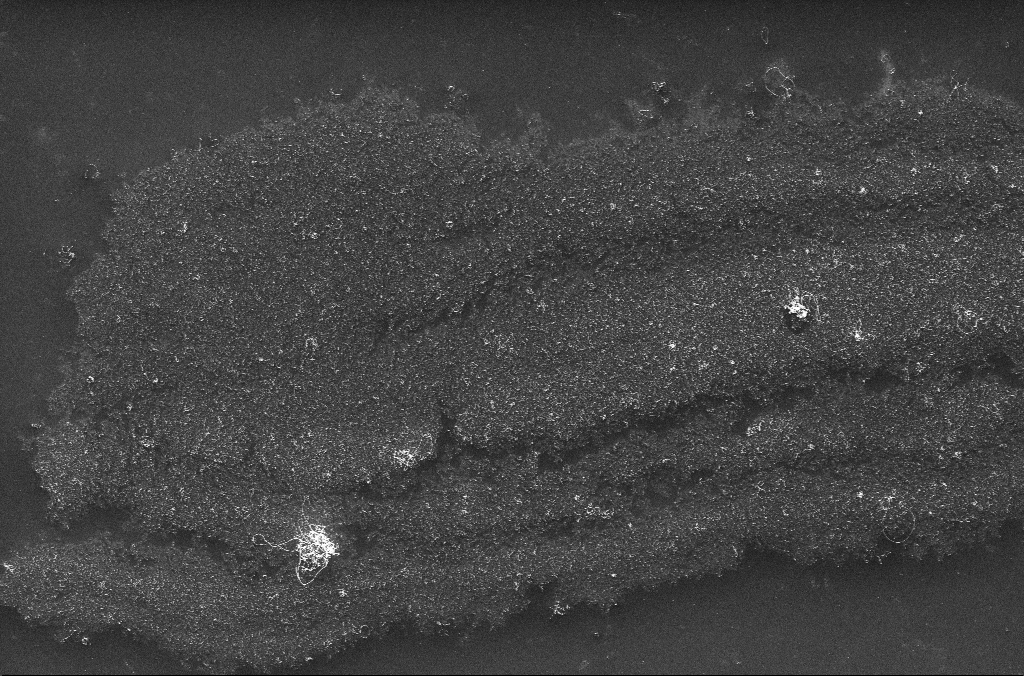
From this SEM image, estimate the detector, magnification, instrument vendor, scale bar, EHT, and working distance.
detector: InLens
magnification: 6.14 K X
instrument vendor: Zeiss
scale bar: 10000 nm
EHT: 10 kV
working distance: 3.2 mm